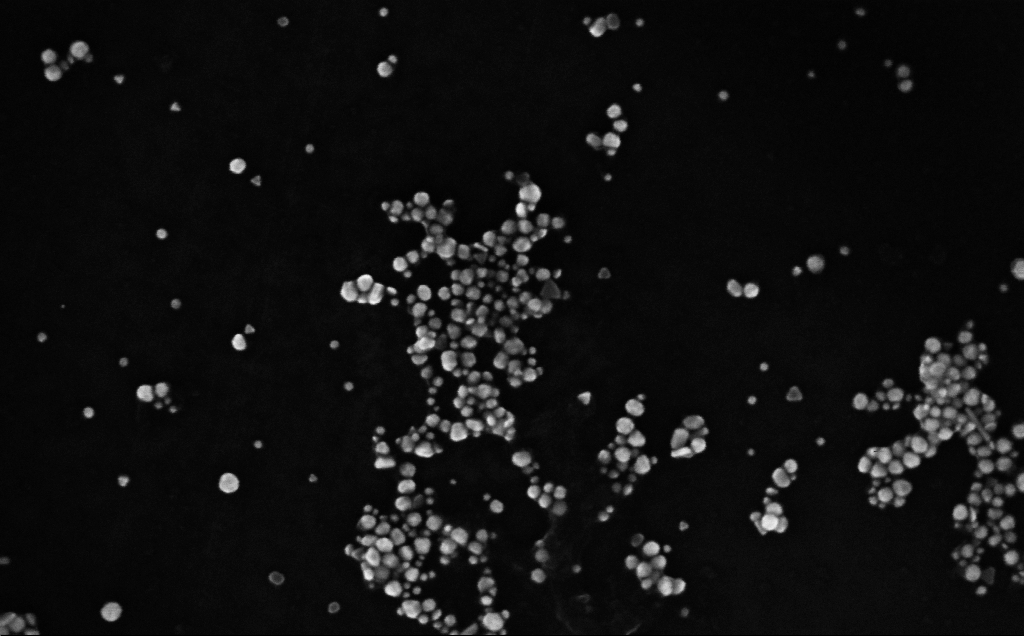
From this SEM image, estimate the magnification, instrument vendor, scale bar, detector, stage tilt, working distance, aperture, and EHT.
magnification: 240.78 K X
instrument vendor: Zeiss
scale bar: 100 nm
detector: InLens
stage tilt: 0°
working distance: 3 mm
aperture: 30 µm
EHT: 10 kV